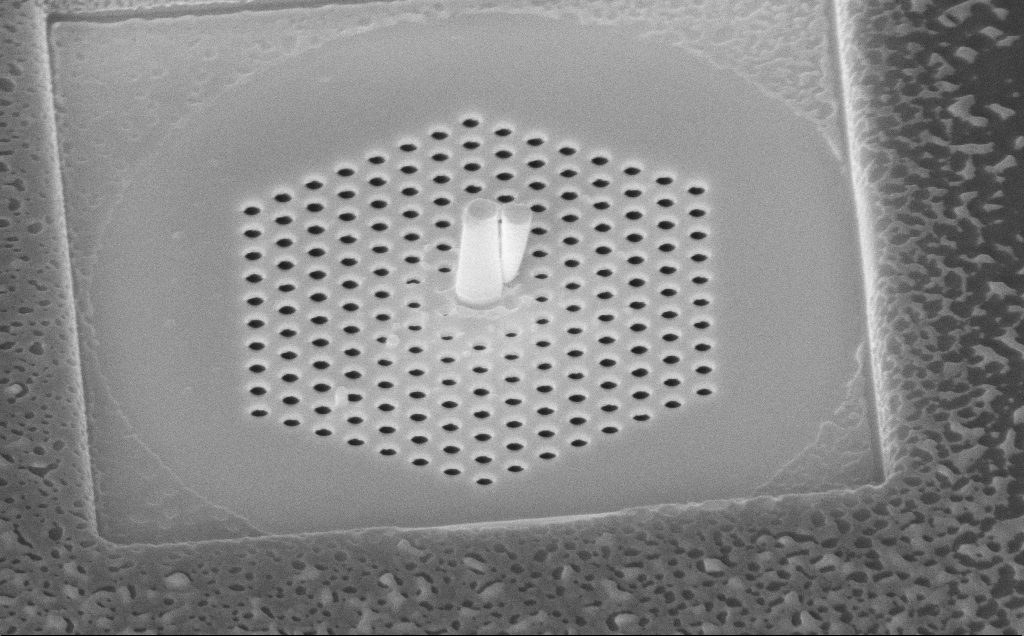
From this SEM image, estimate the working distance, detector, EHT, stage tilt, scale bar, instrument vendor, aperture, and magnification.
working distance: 11 mm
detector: InLens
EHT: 10 kV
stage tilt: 45°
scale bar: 1000 nm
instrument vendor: Zeiss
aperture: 30 µm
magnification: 46.81 K X